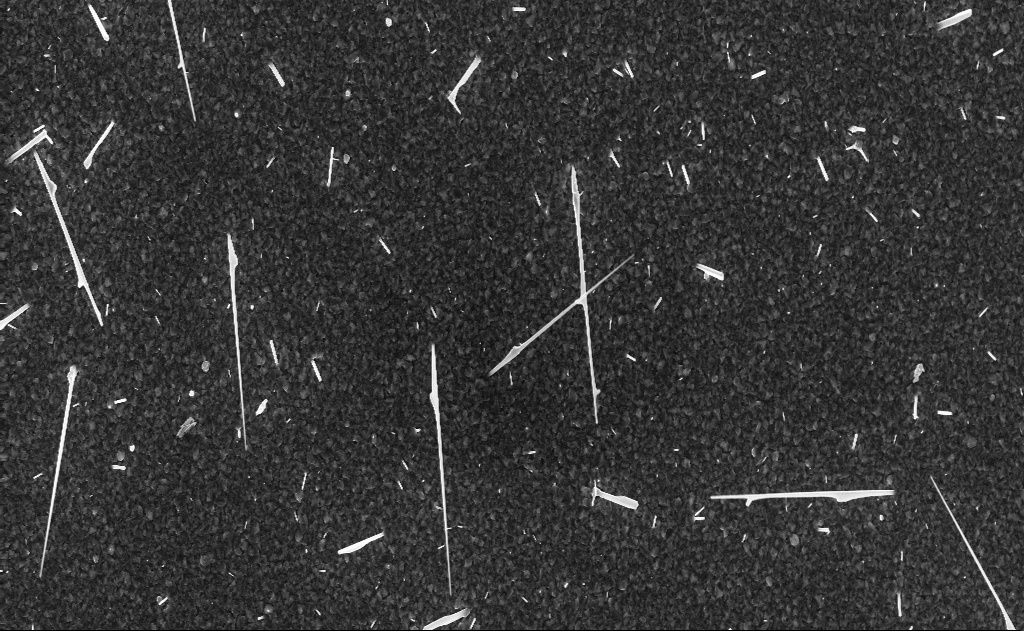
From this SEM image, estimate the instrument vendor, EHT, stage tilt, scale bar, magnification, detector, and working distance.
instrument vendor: Zeiss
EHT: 10 kV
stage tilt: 0°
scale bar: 10000 nm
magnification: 5 K X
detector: InLens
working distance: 6 mm